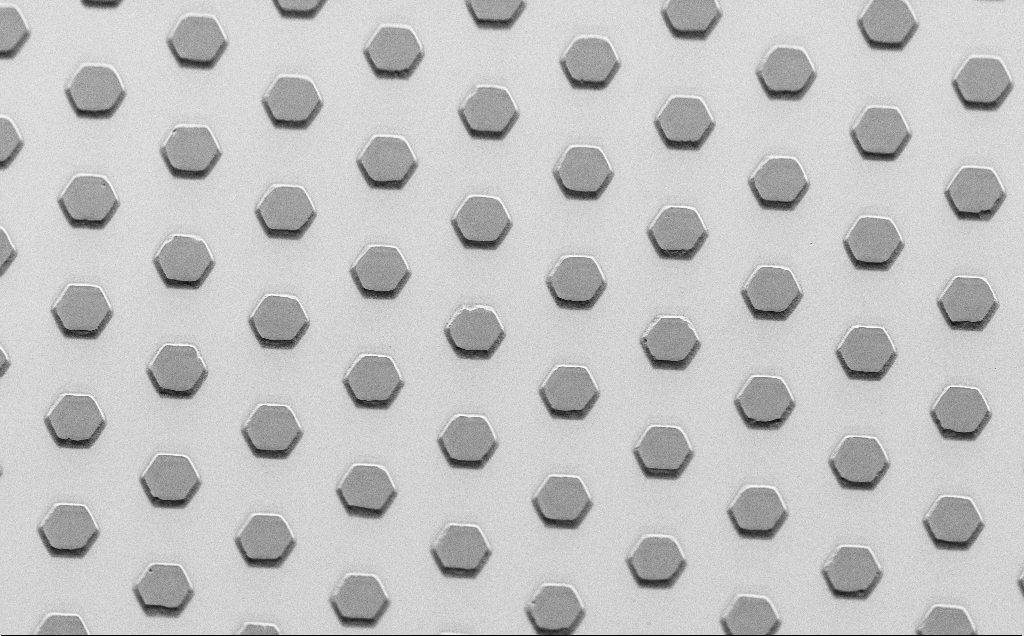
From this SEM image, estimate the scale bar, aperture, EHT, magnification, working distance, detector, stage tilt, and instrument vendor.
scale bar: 20000 nm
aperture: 30 µm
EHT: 1.5 kV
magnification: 1.04 K X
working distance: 7 mm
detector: SE2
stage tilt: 45°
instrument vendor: Zeiss